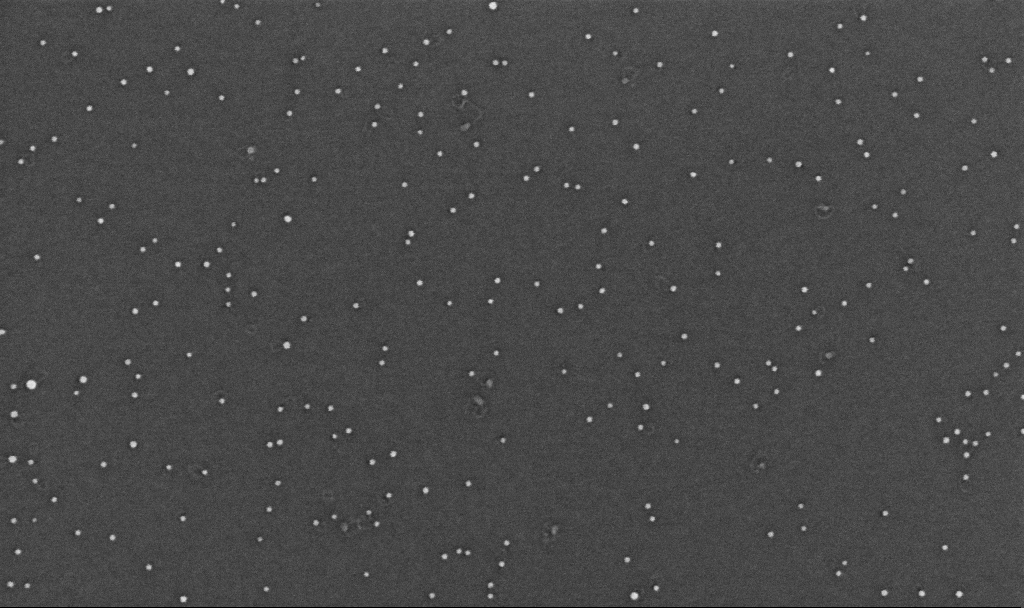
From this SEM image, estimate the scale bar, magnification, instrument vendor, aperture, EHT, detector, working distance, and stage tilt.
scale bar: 100 nm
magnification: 200 K X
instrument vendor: Zeiss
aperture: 30 µm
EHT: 10 kV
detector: InLens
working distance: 3.3 mm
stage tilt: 0°